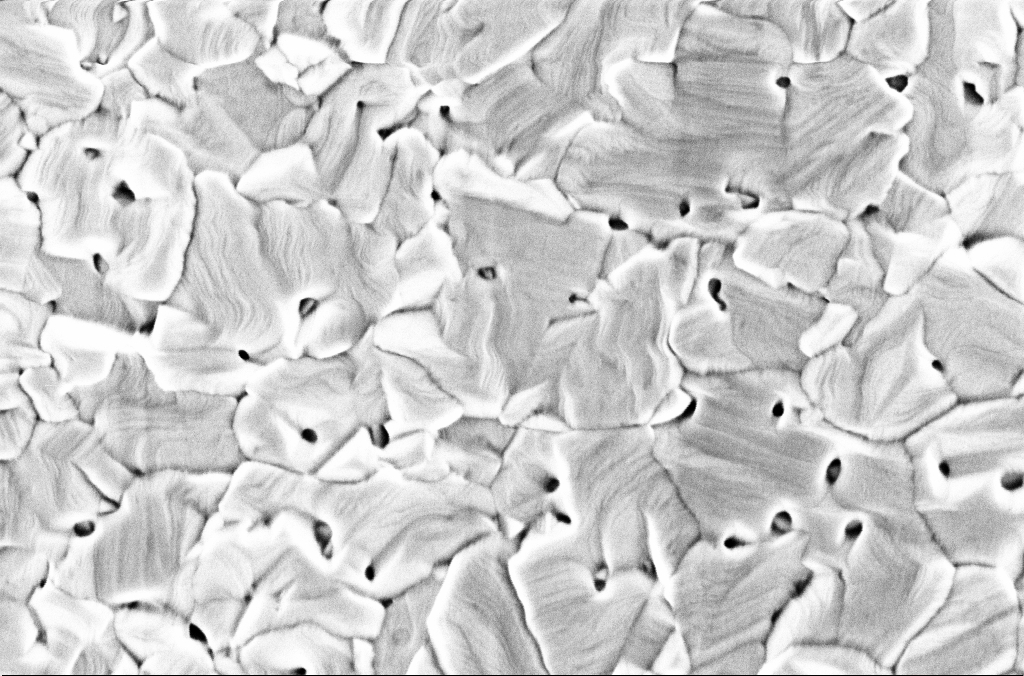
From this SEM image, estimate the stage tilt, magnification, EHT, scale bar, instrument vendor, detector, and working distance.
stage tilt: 0°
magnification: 80 K X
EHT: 2 kV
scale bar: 200 nm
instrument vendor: Zeiss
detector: InLens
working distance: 3 mm